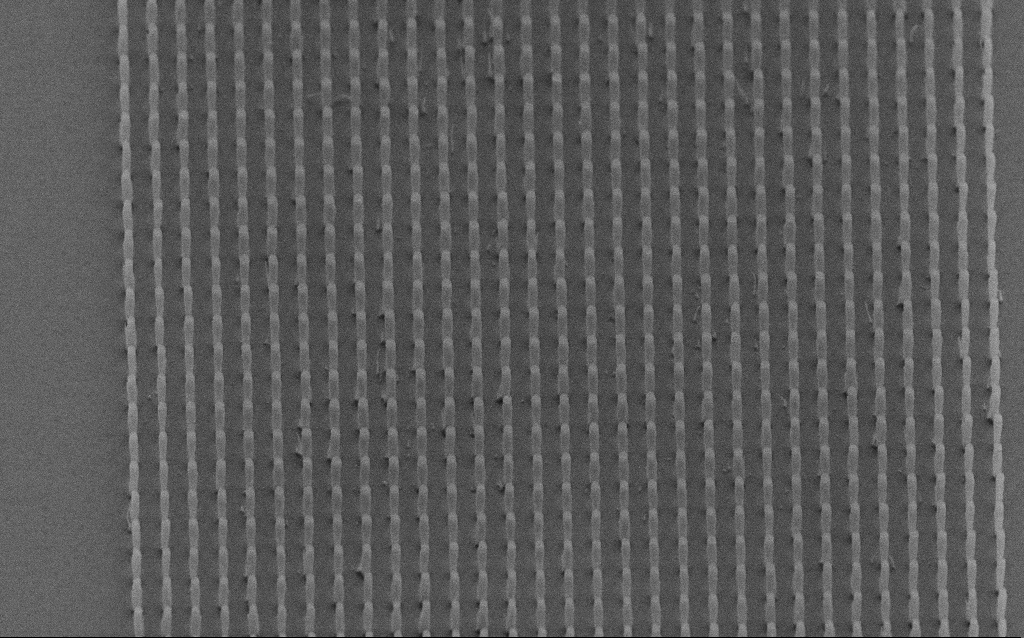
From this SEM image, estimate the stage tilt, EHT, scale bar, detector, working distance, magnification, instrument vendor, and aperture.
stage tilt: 45°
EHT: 5 kV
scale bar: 1000 nm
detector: SE2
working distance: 7 mm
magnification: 13.16 K X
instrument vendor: Zeiss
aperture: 30 µm